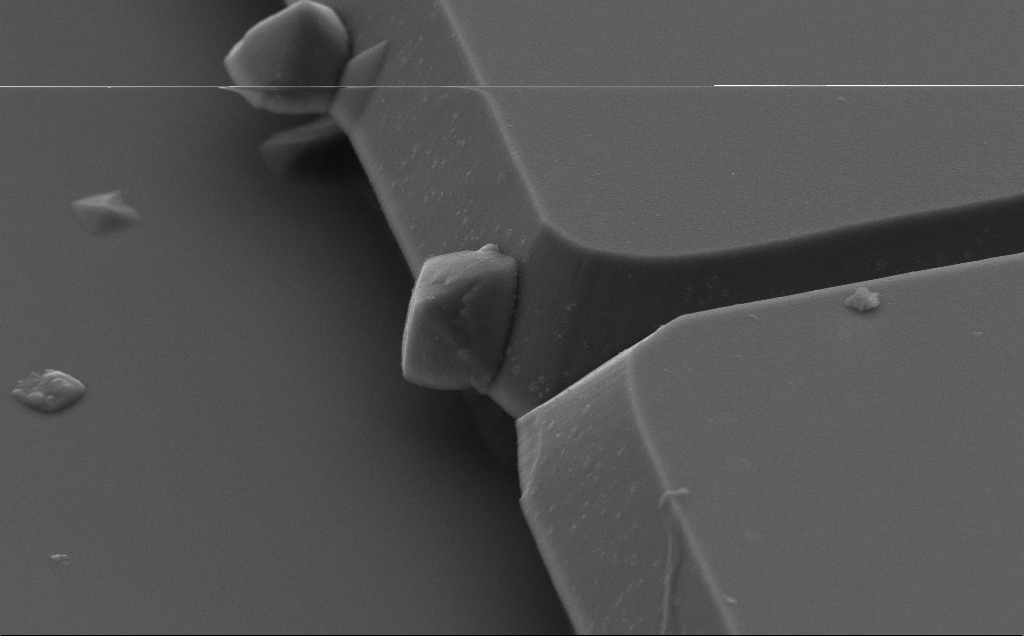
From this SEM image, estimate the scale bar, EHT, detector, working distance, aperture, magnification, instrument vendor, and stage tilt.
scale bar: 2000 nm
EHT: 5 kV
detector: SE2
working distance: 10 mm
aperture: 30 µm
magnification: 20.17 K X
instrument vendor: Zeiss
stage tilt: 50°